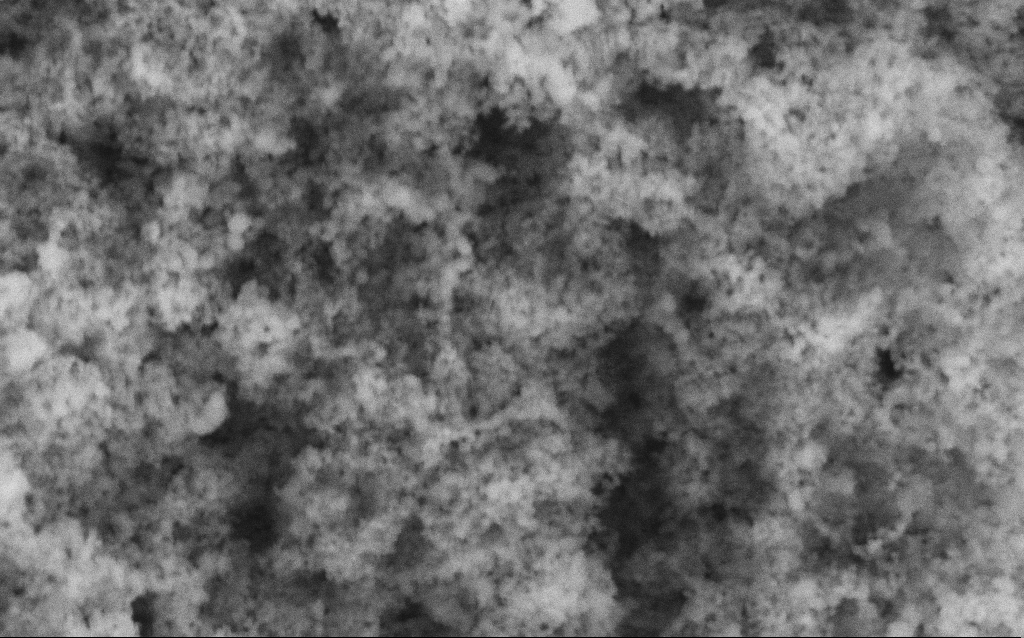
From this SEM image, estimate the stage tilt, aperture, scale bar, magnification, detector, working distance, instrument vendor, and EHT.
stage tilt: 0°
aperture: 30 µm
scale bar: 200 nm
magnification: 114.64 K X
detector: SE2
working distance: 4.2 mm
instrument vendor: Zeiss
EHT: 5 kV